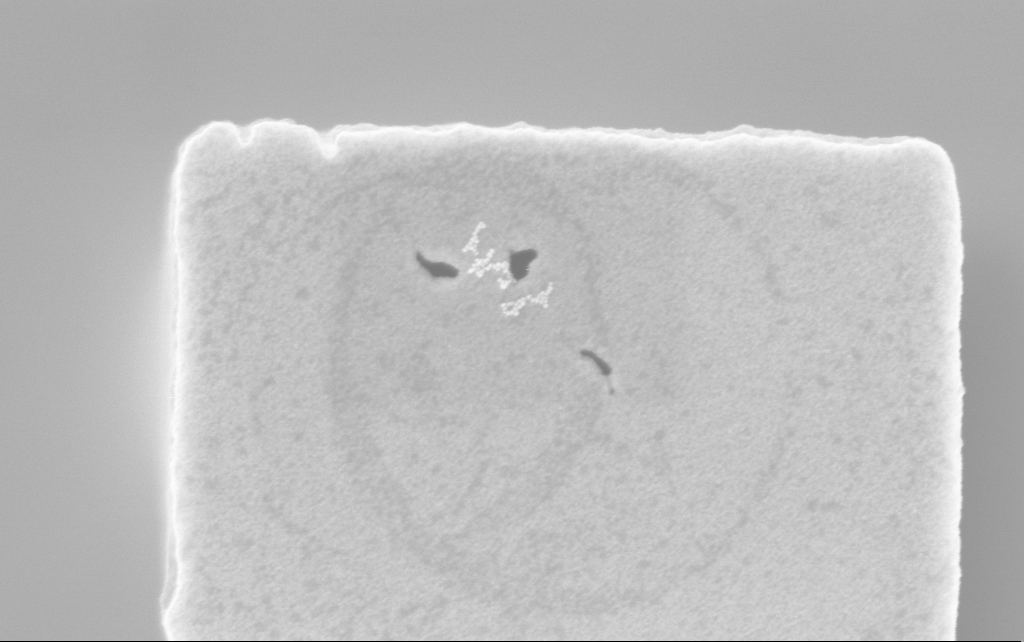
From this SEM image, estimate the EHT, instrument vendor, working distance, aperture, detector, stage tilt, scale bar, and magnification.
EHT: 3 kV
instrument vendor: Zeiss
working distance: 2.5 mm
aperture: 30 µm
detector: InLens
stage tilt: -0°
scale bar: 200 nm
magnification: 96.28 K X